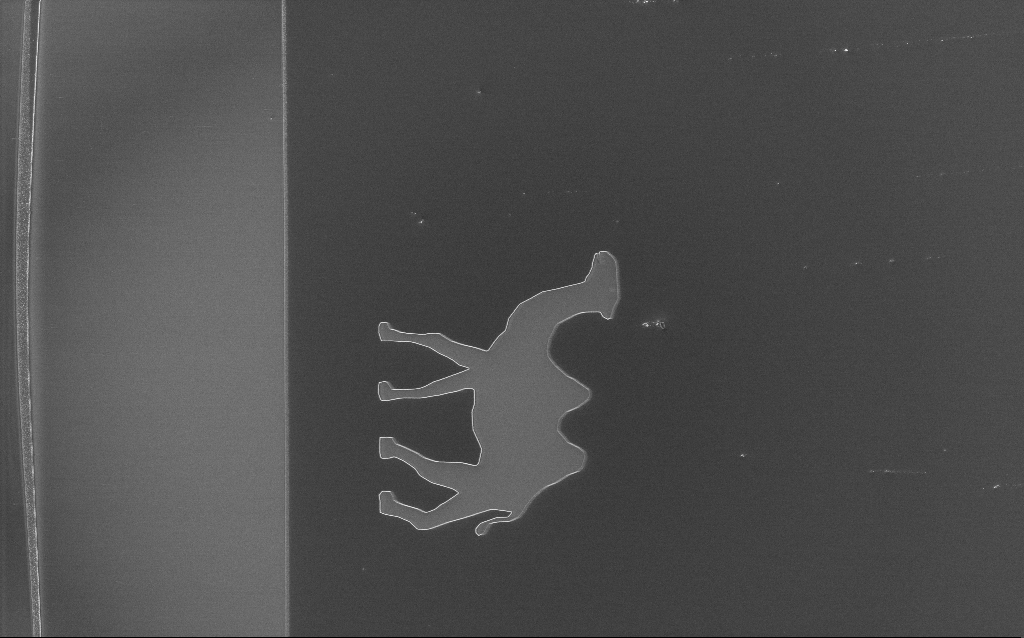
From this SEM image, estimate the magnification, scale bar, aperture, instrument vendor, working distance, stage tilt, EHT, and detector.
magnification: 0.405 K X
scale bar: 100000 nm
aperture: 30 µm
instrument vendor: Zeiss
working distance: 5 mm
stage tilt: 0°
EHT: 3 kV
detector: InLens